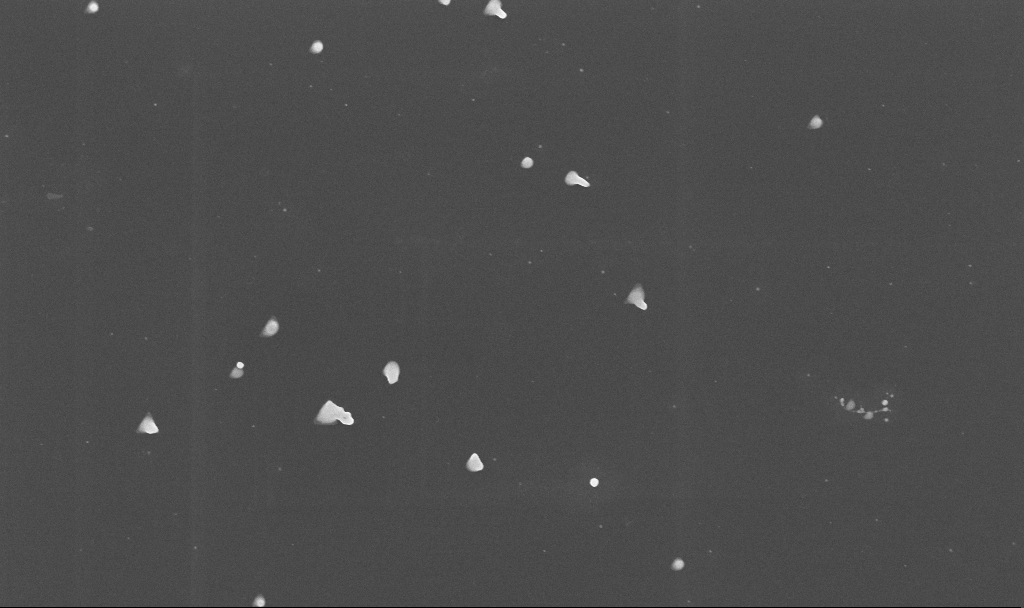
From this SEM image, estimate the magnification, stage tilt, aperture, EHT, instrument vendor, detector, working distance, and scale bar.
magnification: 50 K X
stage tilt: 0°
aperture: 30 µm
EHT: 10 kV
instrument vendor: Zeiss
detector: InLens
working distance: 3.4 mm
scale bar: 1000 nm